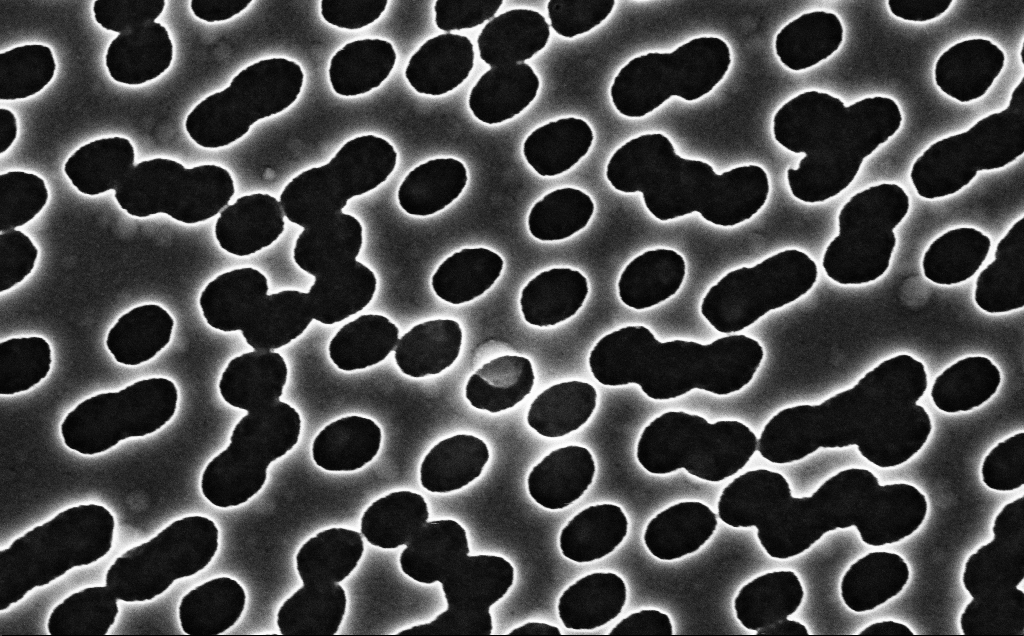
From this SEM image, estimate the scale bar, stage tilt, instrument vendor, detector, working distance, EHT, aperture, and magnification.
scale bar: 200 nm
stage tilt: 0°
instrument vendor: Zeiss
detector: InLens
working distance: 2.5 mm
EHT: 3 kV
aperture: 30 µm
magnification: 90 K X